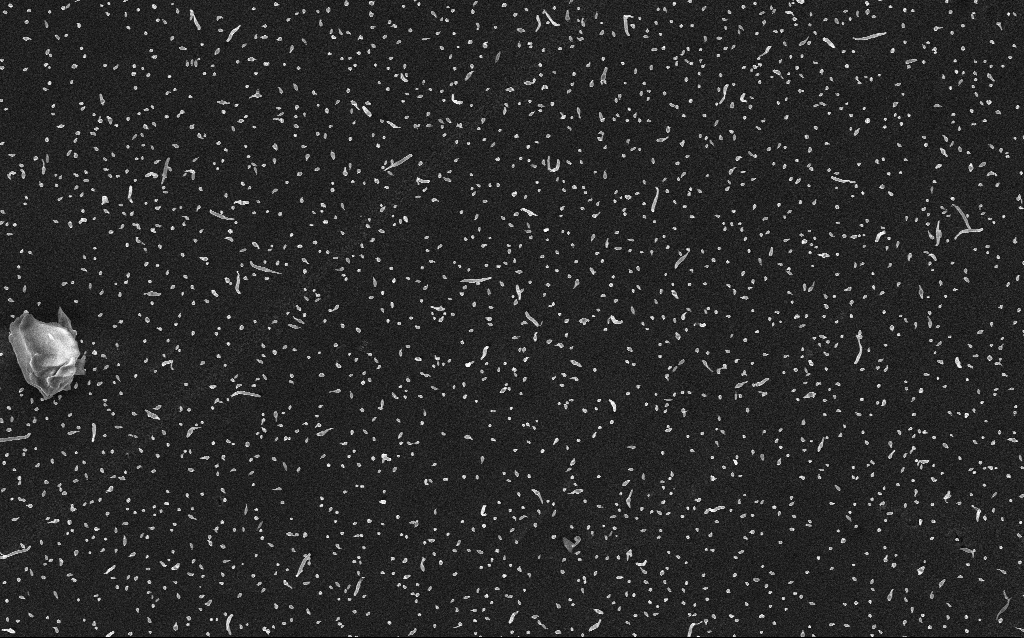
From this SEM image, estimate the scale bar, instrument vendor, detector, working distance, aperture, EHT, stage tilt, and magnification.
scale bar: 2000 nm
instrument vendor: Zeiss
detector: InLens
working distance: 2.1 mm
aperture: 30 µm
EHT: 5 kV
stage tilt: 0°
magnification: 10 K X